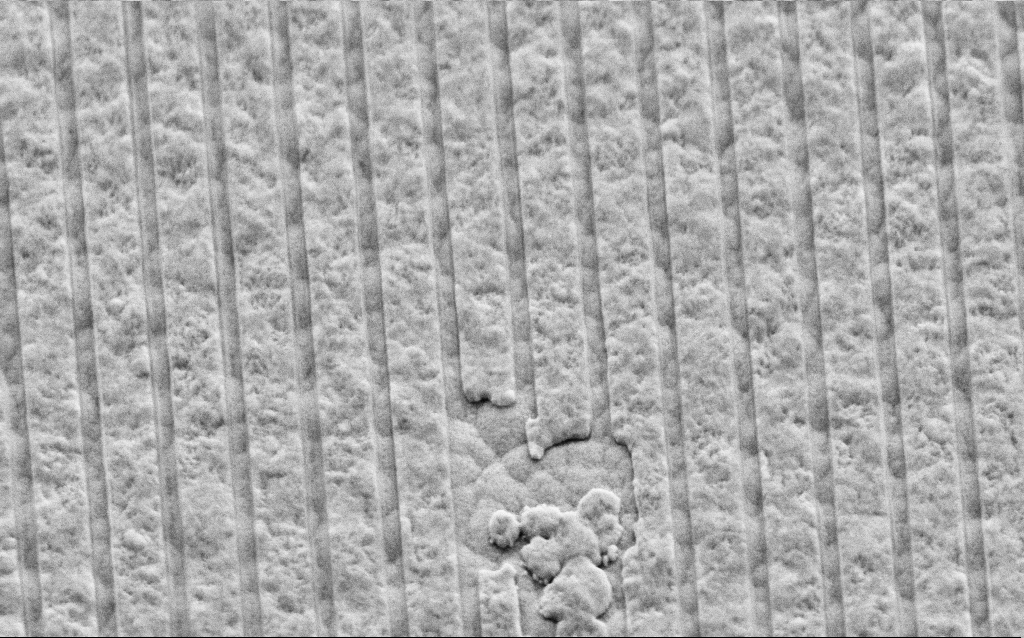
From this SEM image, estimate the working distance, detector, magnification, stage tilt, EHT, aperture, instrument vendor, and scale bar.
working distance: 6 mm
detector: SE2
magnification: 53.76 K X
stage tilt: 45°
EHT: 3 kV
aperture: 30 µm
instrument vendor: Zeiss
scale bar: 1000 nm